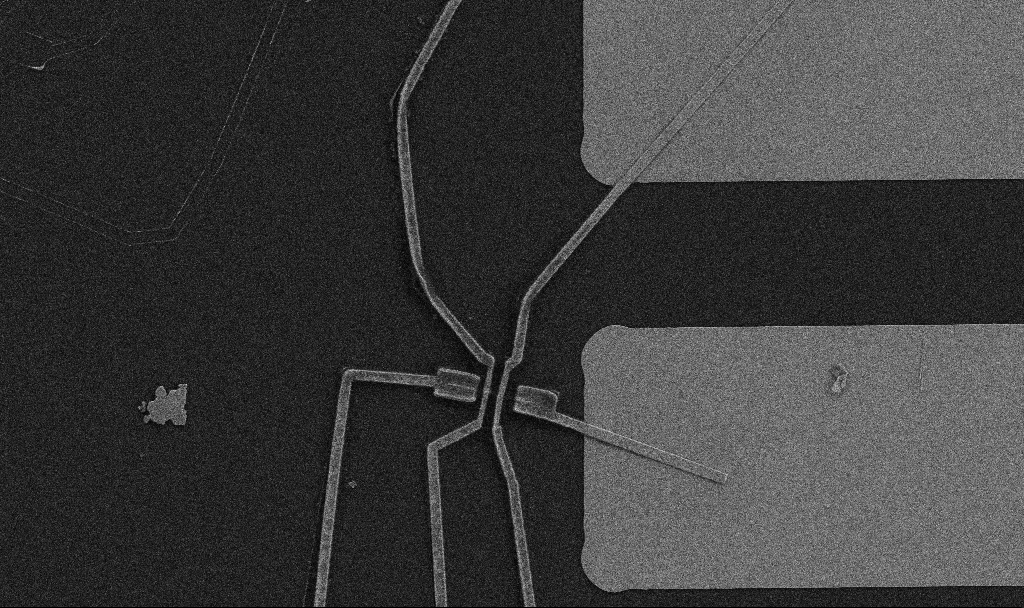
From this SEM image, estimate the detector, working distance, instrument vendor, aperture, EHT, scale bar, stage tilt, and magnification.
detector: SE2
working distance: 9.7 mm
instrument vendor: Zeiss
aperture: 30 µm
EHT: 5 kV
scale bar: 10000 nm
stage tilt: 0°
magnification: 5 K X